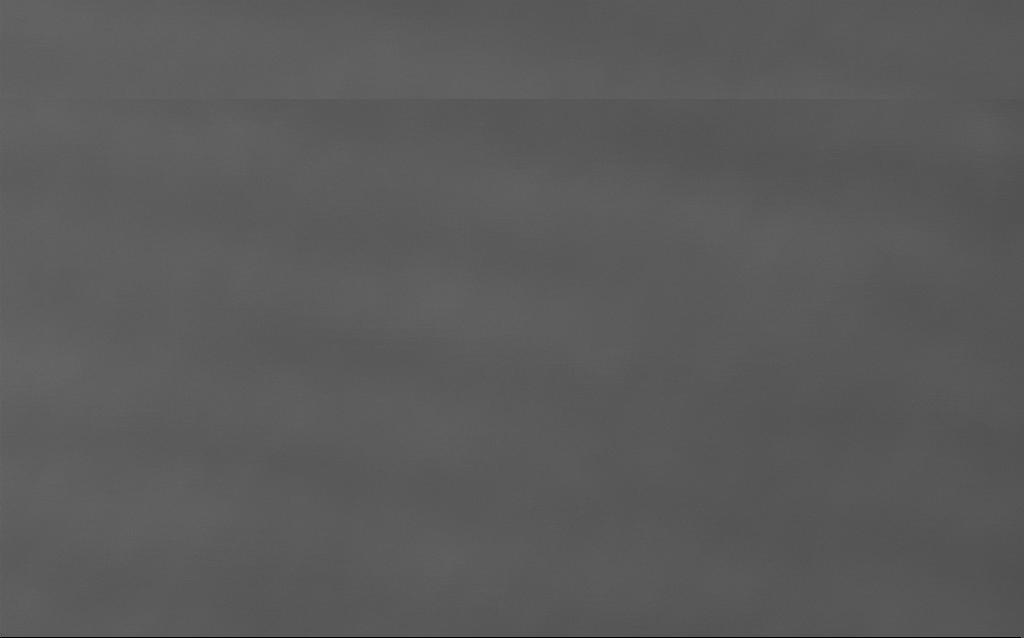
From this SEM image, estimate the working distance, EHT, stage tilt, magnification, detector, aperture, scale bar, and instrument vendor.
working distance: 4 mm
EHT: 5 kV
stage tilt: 0°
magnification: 3204.55 K X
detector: InLens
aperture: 30 µm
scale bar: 20 nm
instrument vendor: Zeiss